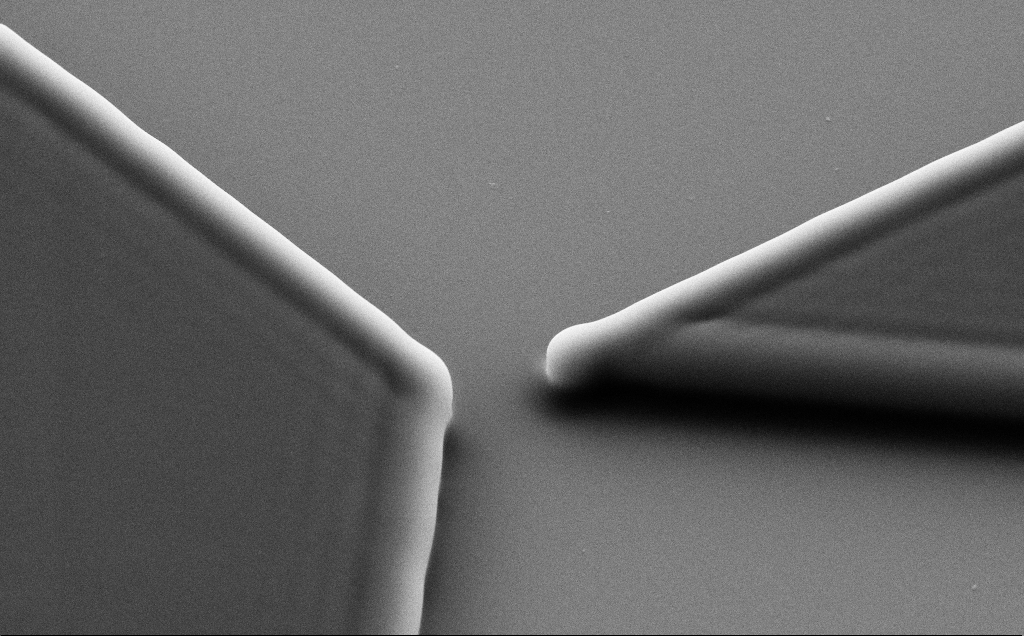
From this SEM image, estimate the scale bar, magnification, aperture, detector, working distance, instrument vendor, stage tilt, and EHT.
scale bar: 1000 nm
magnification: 16.78 K X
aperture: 30 µm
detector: SE2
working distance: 5 mm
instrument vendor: Zeiss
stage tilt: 35°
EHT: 7 kV